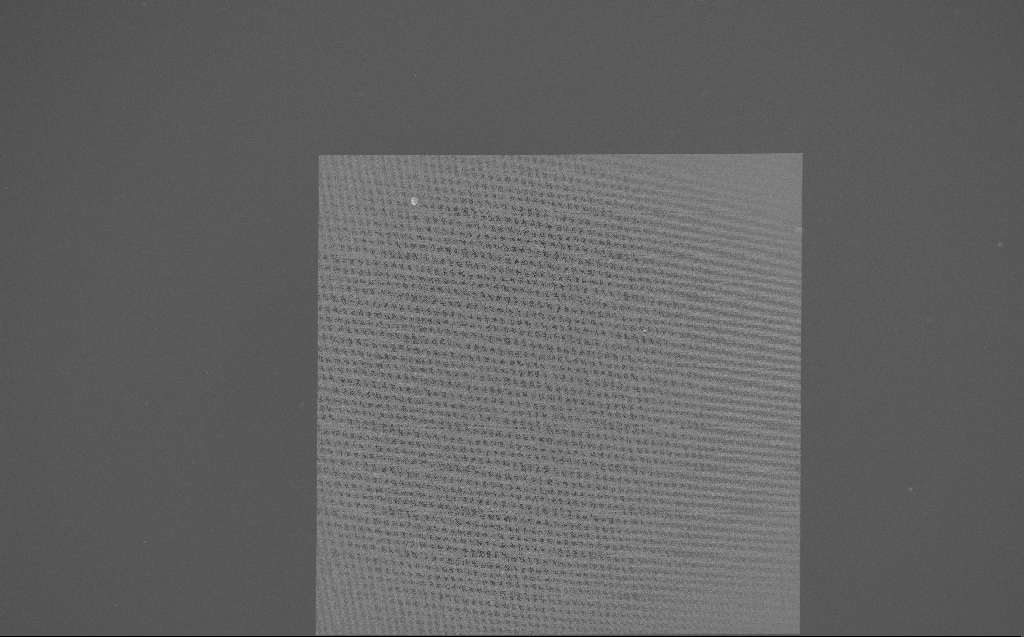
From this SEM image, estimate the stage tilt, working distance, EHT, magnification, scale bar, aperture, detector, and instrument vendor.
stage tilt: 0°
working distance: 7 mm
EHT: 5 kV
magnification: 0.238 K X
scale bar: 100000 nm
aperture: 30 µm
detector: InLens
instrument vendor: Zeiss